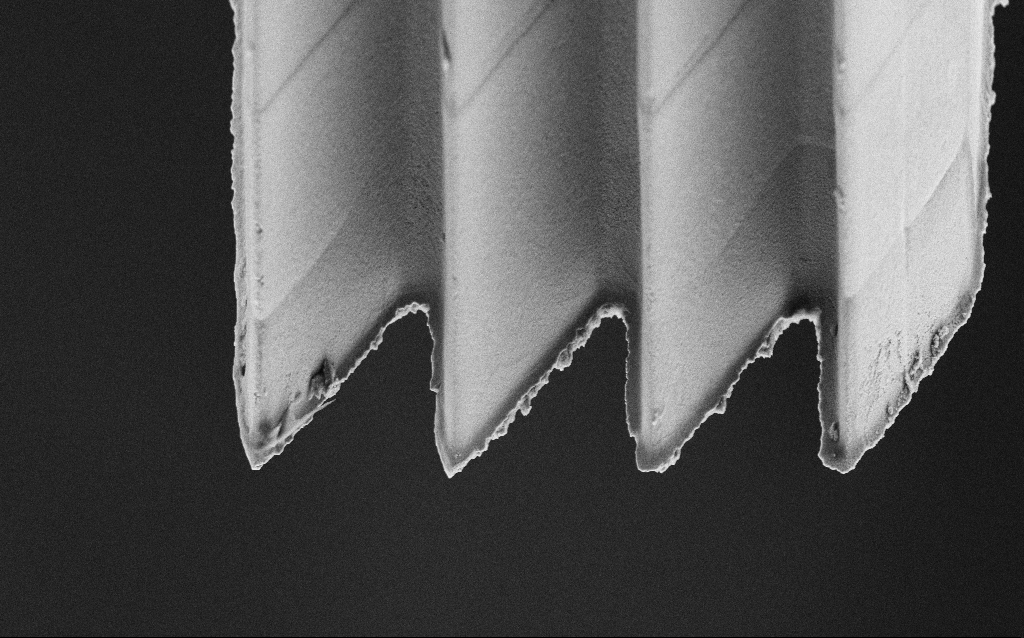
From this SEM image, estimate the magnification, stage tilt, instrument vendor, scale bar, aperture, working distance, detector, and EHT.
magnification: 6.39 K X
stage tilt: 45°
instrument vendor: Zeiss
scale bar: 10000 nm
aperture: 30 µm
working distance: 7 mm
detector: SE2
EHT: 5 kV